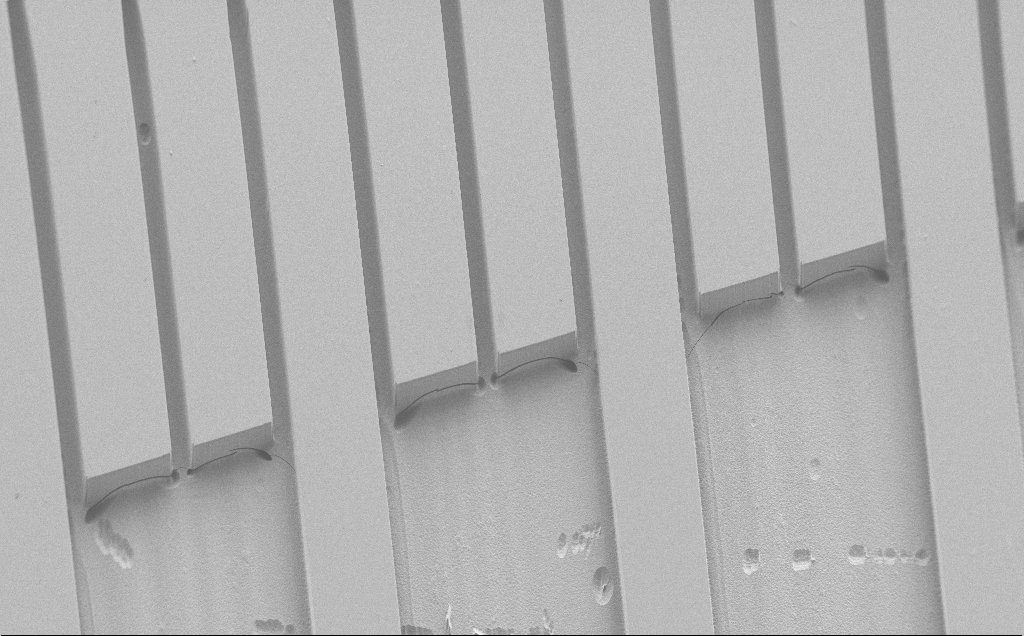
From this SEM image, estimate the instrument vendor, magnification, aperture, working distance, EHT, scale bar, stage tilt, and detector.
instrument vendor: Zeiss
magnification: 0.354 K X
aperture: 30 µm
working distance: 7 mm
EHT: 1.2 kV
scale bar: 100000 nm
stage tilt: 30°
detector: SE2